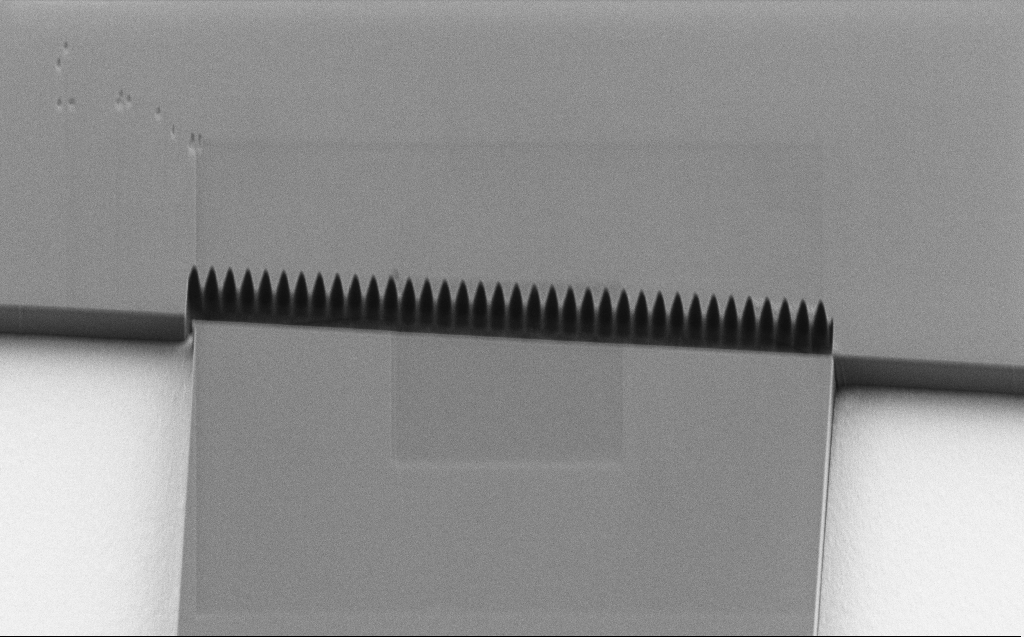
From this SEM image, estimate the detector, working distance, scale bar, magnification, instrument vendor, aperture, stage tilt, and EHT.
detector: SE2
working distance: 6 mm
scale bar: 2000 nm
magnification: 7.43 K X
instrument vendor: Zeiss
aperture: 30 µm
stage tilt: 30°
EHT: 1.1 kV